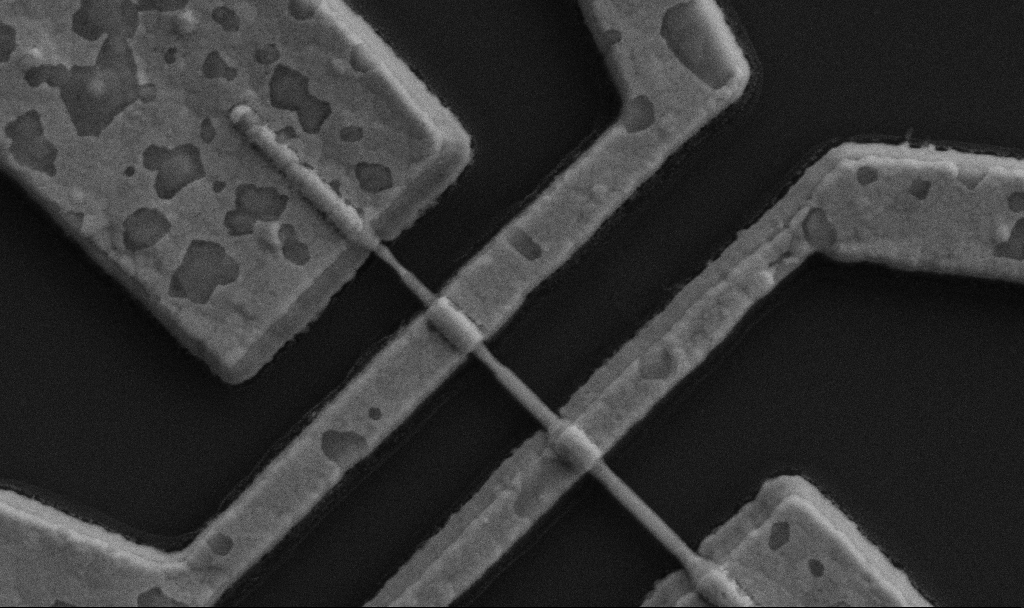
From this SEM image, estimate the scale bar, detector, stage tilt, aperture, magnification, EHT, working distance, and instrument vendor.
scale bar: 1000 nm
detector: SE2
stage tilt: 0°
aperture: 30 µm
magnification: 60 K X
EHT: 5 kV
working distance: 9.7 mm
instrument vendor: Zeiss